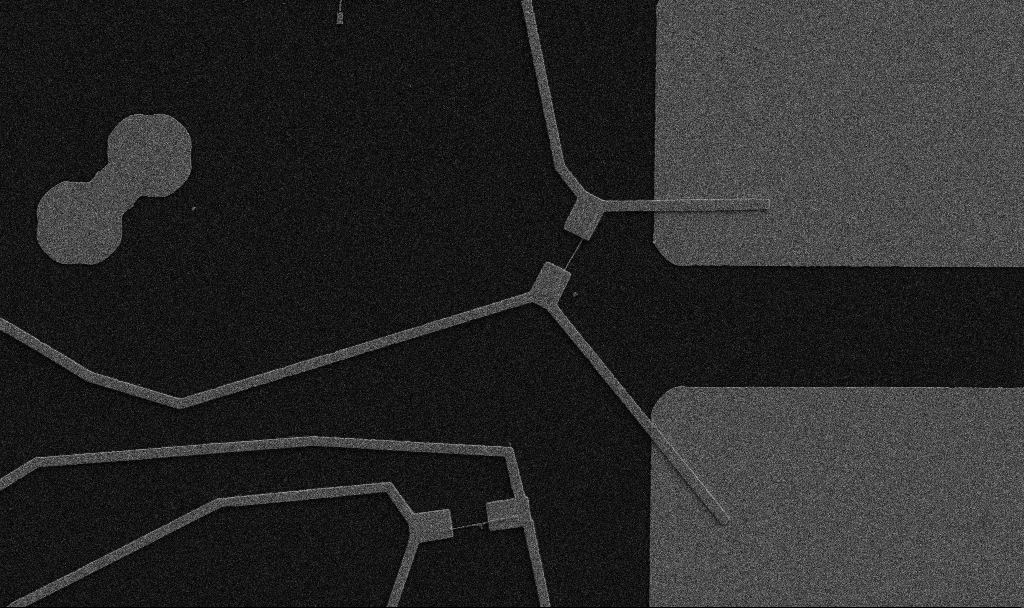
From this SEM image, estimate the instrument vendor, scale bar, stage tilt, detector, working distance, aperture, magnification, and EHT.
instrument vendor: Zeiss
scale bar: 10000 nm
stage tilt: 0°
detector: SE2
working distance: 10.7 mm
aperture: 30 µm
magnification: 5 K X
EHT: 5 kV